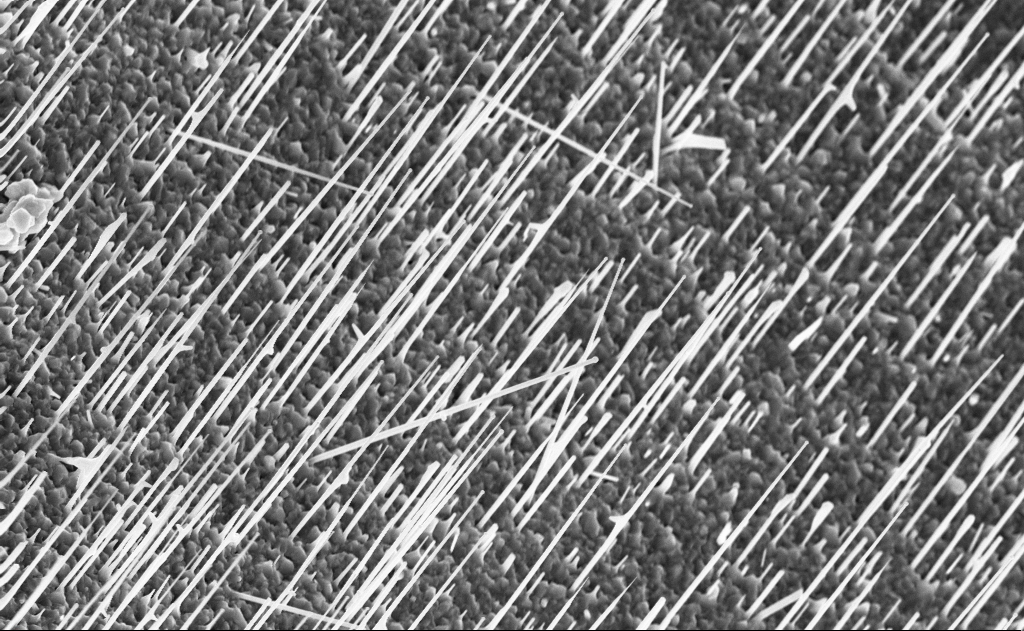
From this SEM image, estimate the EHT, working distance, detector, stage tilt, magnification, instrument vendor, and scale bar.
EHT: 10 kV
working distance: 10 mm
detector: InLens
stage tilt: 0°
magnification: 15 K X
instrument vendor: Zeiss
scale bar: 2000 nm